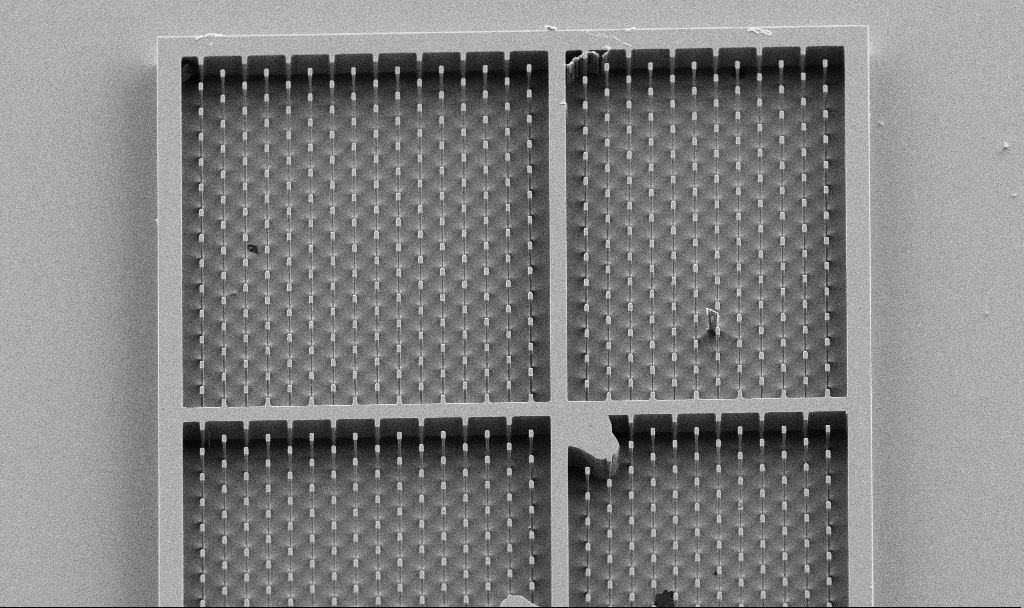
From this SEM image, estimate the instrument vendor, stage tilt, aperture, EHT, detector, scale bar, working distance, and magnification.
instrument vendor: Zeiss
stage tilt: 29.2°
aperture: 30 µm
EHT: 5 kV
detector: SE2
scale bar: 20000 nm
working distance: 6.9 mm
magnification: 0.896 K X